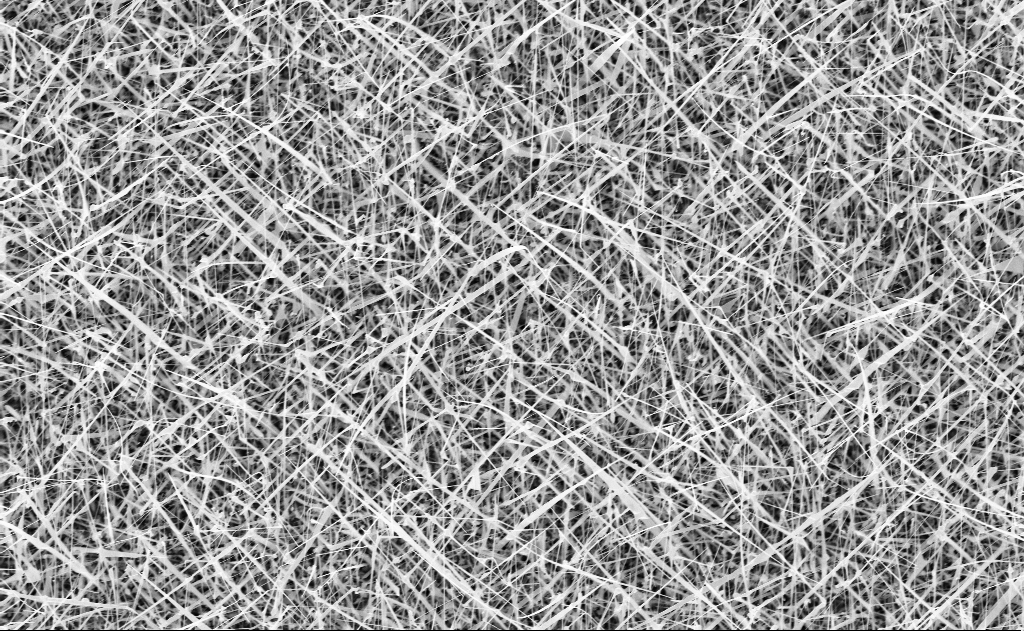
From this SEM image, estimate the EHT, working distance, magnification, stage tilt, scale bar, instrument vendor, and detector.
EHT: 10 kV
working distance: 10 mm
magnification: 10 K X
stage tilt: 0°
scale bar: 2000 nm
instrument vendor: Zeiss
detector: InLens